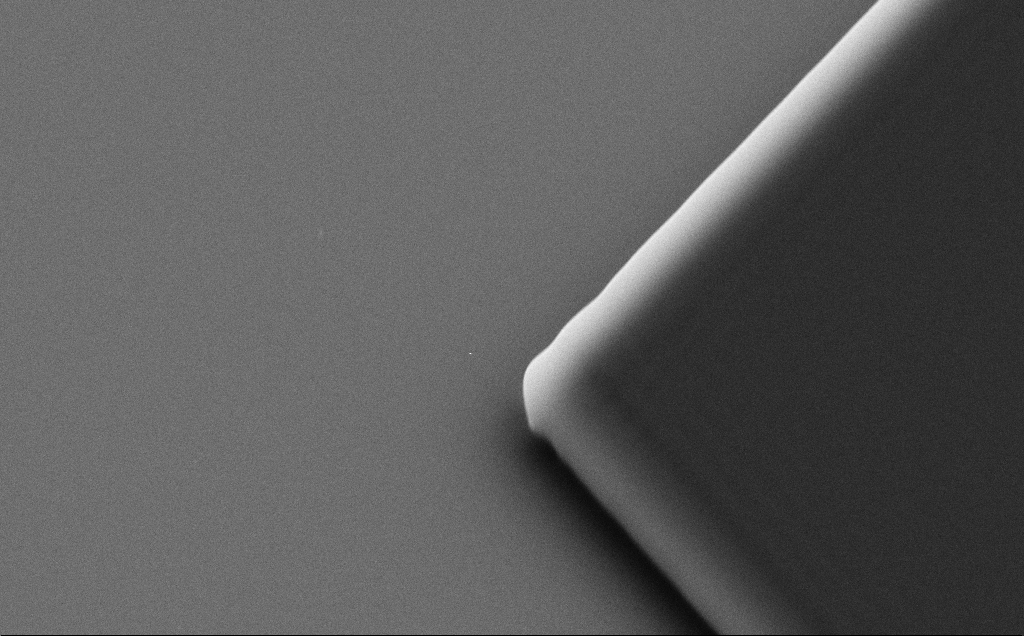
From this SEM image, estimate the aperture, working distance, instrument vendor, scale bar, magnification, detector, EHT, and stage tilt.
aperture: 30 µm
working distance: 8 mm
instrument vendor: Zeiss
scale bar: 2000 nm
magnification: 14.82 K X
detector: SE2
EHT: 10 kV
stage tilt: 35°